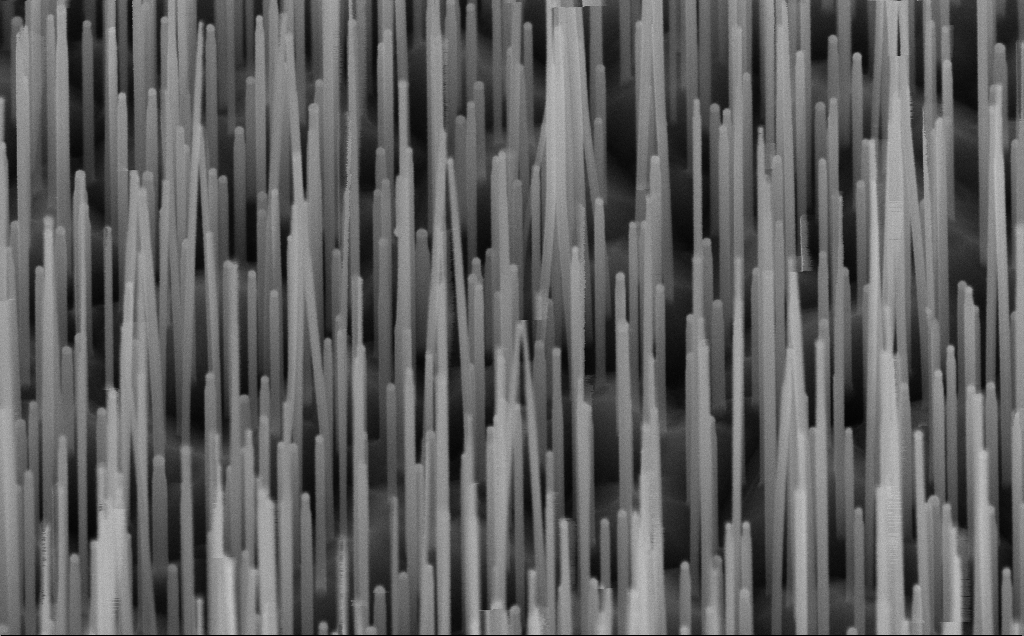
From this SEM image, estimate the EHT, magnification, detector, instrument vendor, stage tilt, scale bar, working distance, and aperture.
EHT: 10 kV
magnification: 100 K X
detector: InLens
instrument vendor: Zeiss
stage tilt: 45°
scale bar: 200 nm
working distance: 7 mm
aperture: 30 µm